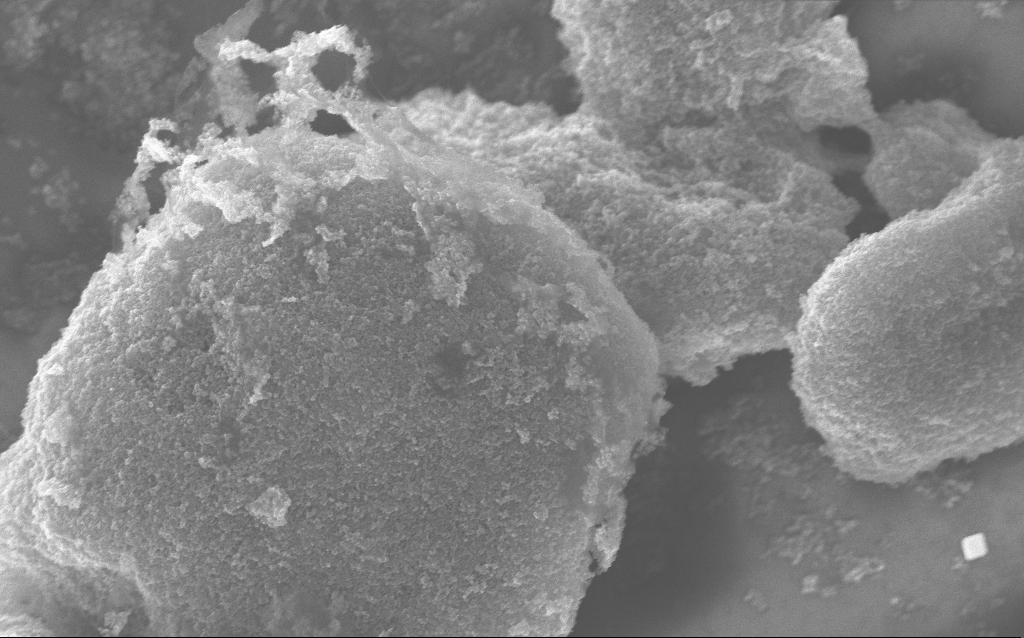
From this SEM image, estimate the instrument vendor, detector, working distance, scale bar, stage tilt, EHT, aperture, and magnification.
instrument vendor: Zeiss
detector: InLens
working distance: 2.8 mm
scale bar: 1000 nm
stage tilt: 0°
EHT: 10 kV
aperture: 30 µm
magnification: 37.88 K X